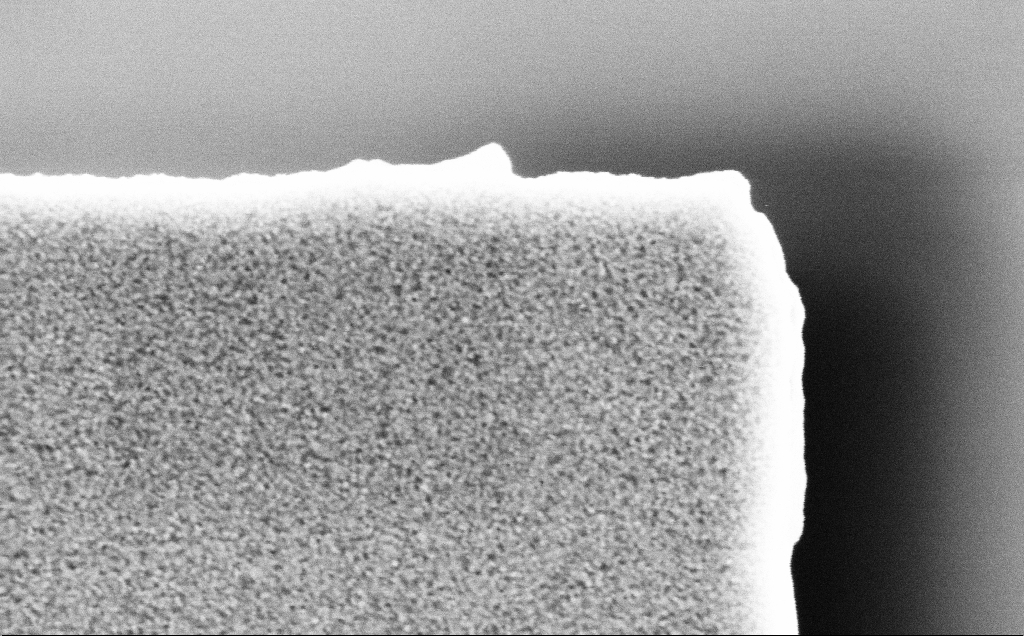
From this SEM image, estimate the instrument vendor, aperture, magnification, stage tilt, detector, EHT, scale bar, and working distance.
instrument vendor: Zeiss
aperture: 30 µm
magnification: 180.38 K X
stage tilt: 0°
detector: InLens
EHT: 3 kV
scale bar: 200 nm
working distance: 3.1 mm